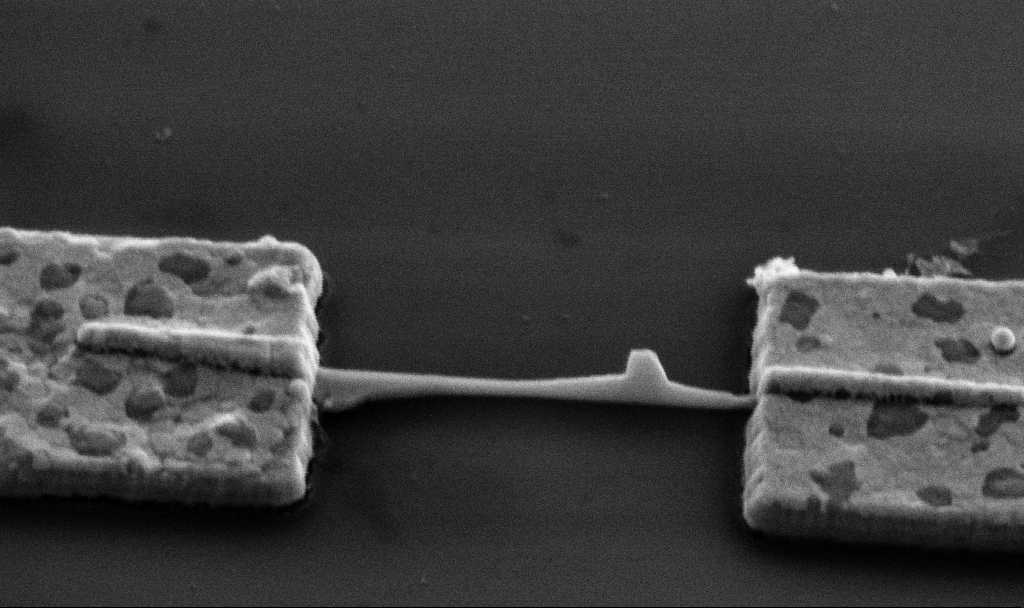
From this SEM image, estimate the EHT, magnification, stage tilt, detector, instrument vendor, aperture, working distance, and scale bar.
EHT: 5 kV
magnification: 60 K X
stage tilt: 45°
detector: SE2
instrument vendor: Zeiss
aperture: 30 µm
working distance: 13.6 mm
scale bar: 1000 nm